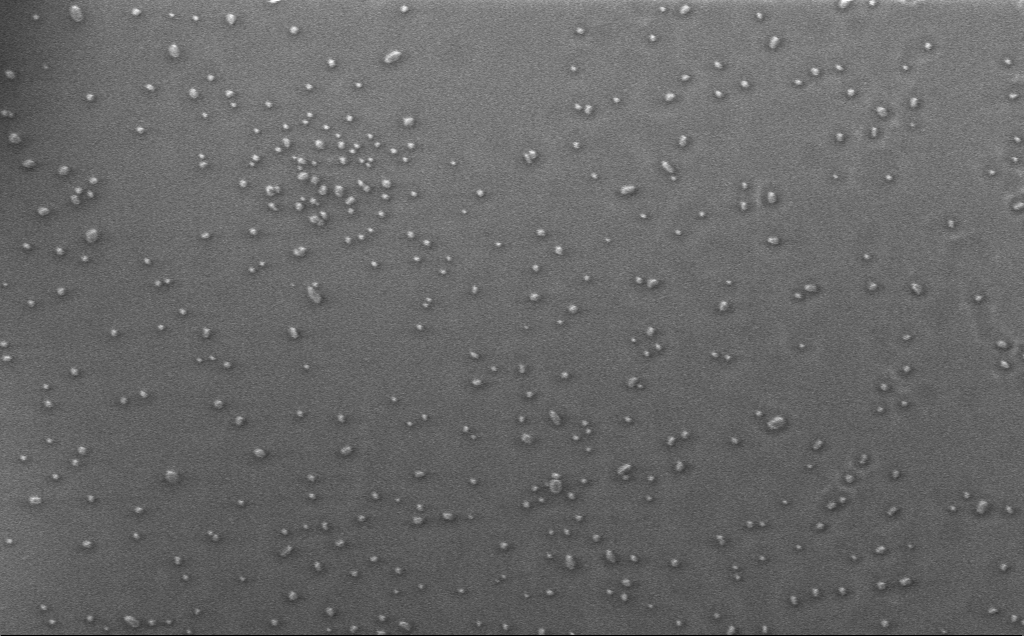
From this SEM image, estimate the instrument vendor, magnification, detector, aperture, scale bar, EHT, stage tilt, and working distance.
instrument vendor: Zeiss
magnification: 7.14 K X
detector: InLens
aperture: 30 µm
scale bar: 10000 nm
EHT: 1 kV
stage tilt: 0°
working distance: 3 mm